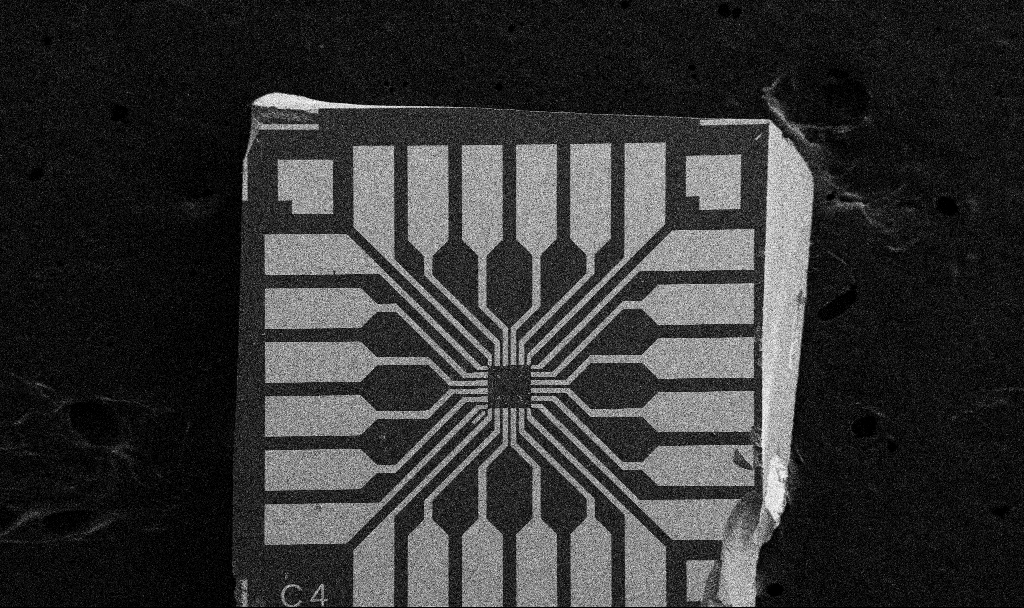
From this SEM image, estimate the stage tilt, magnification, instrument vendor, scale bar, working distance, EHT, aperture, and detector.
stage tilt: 0°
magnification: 0.1 K X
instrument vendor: Zeiss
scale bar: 200000 nm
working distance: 8.7 mm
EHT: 5 kV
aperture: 30 µm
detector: SE2